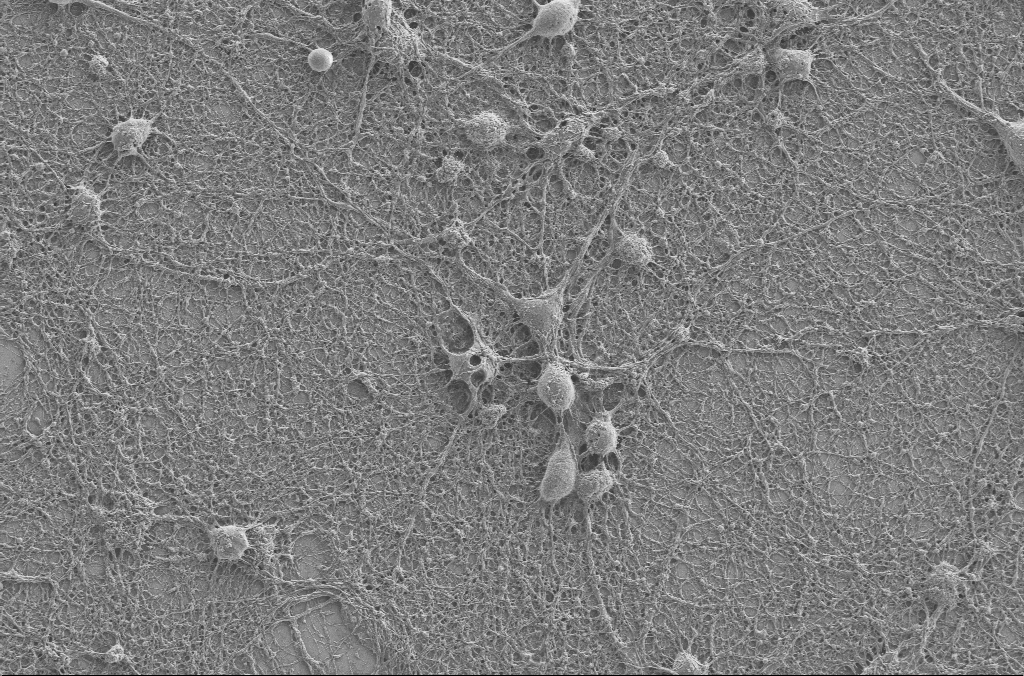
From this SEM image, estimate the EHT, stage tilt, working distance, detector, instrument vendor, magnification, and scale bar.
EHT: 2 kV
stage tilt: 0°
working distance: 4 mm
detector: SE2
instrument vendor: Zeiss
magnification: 2.5 K X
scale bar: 20000 nm